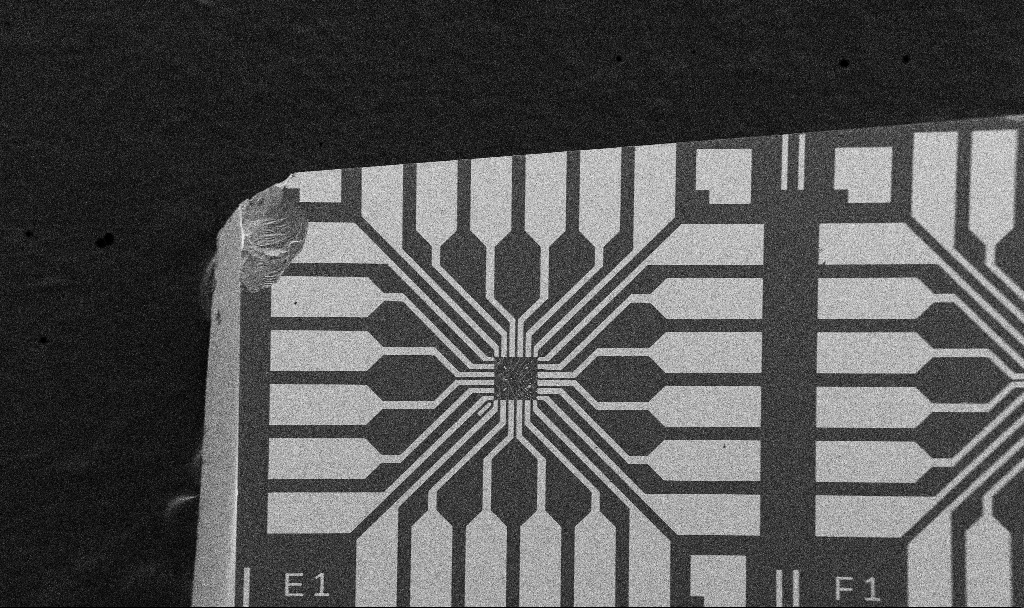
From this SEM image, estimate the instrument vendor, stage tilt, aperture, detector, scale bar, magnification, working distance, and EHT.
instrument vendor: Zeiss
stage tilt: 0°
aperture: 30 µm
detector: SE2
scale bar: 200000 nm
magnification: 0.1 K X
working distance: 10.7 mm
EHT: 5 kV